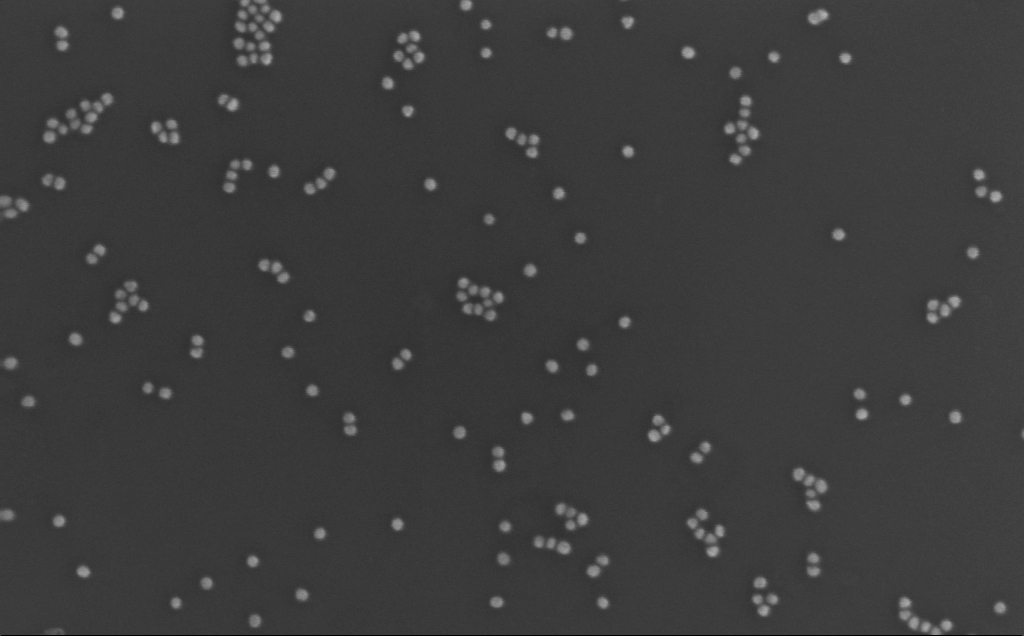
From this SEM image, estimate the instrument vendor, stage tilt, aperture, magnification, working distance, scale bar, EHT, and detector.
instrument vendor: Zeiss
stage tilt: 0°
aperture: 30 µm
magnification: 256.28 K X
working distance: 3 mm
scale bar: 200 nm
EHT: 10 kV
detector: InLens